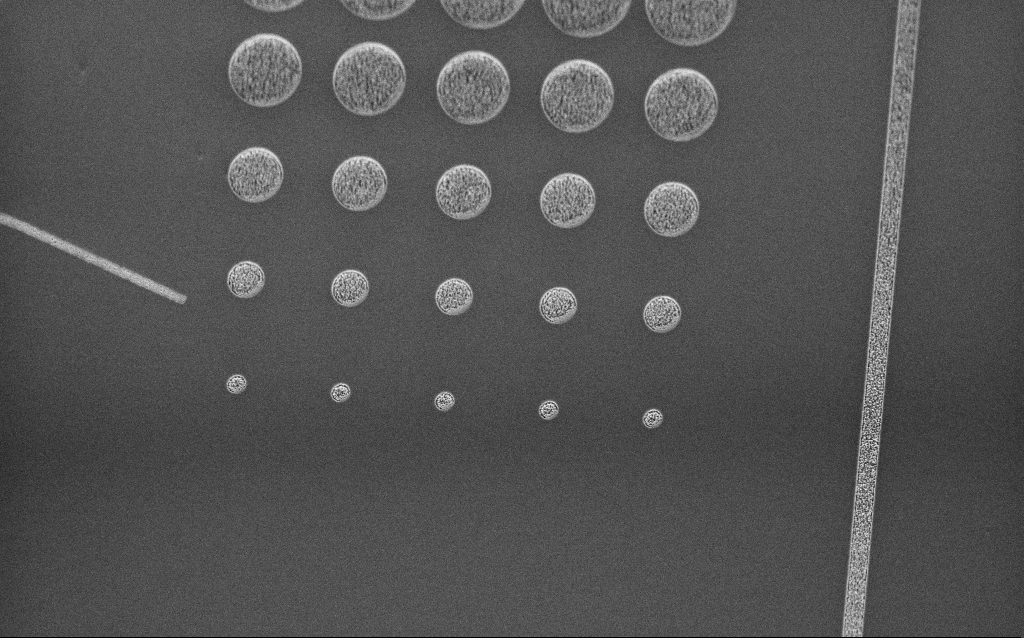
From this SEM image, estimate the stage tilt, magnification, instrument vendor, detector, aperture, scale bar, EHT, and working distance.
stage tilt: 45°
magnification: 6.39 K X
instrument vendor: Zeiss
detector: InLens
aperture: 30 µm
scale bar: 10000 nm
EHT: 3 kV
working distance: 8 mm